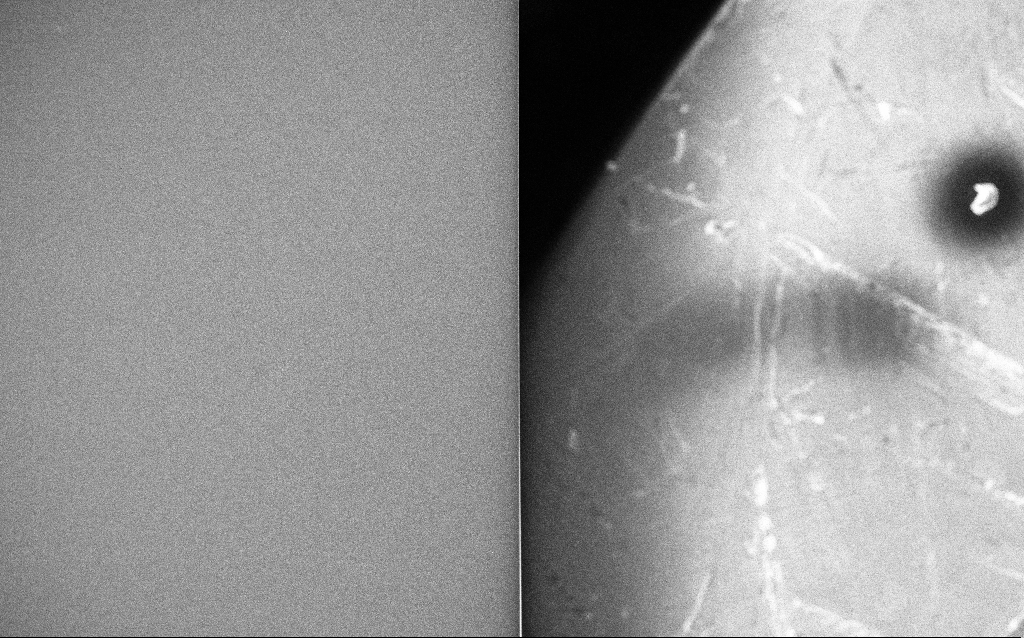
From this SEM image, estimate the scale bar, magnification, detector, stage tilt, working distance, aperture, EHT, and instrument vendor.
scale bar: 100000 nm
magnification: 0.25 K X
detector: InLens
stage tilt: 0°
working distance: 1.8 mm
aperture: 30 µm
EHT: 20 kV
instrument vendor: Zeiss